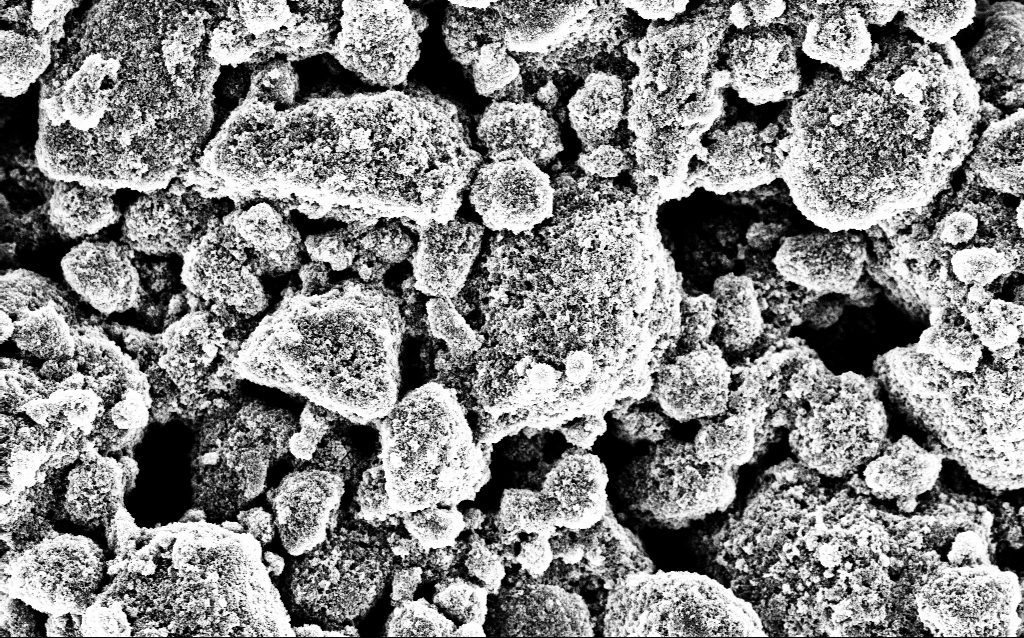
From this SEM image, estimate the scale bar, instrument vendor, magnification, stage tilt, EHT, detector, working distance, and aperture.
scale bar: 2000 nm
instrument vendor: Zeiss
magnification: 16.19 K X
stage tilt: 0°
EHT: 5 kV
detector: InLens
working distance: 1.8 mm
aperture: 30 µm